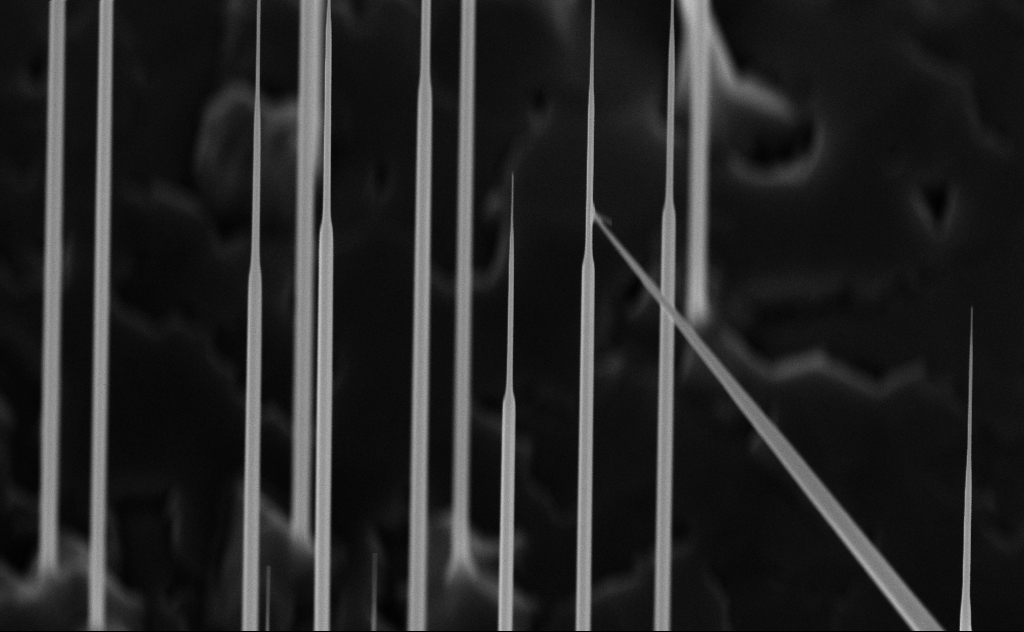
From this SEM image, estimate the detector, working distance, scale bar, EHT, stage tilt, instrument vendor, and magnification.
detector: InLens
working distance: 6 mm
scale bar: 1000 nm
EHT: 10 kV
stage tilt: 45°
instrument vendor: Zeiss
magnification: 40 K X